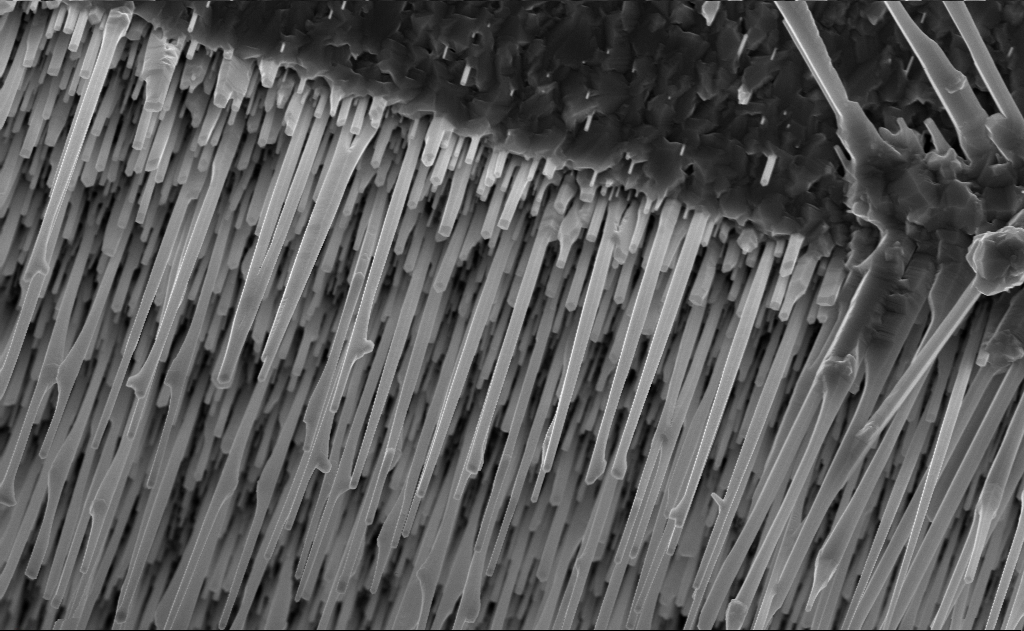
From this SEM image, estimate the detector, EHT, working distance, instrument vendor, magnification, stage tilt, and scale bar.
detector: InLens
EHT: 10 kV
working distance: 9 mm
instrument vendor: Zeiss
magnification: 20 K X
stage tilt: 0°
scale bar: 2000 nm